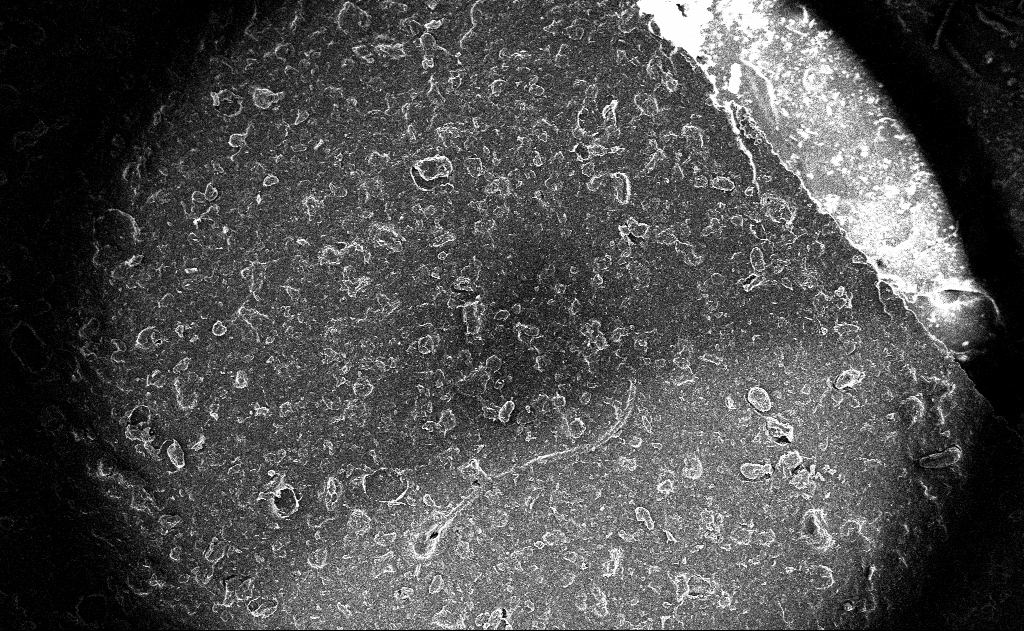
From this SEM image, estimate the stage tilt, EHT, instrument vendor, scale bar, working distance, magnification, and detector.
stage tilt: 0°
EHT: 10 kV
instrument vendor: Zeiss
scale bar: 200000 nm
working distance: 3 mm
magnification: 0.119 K X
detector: InLens